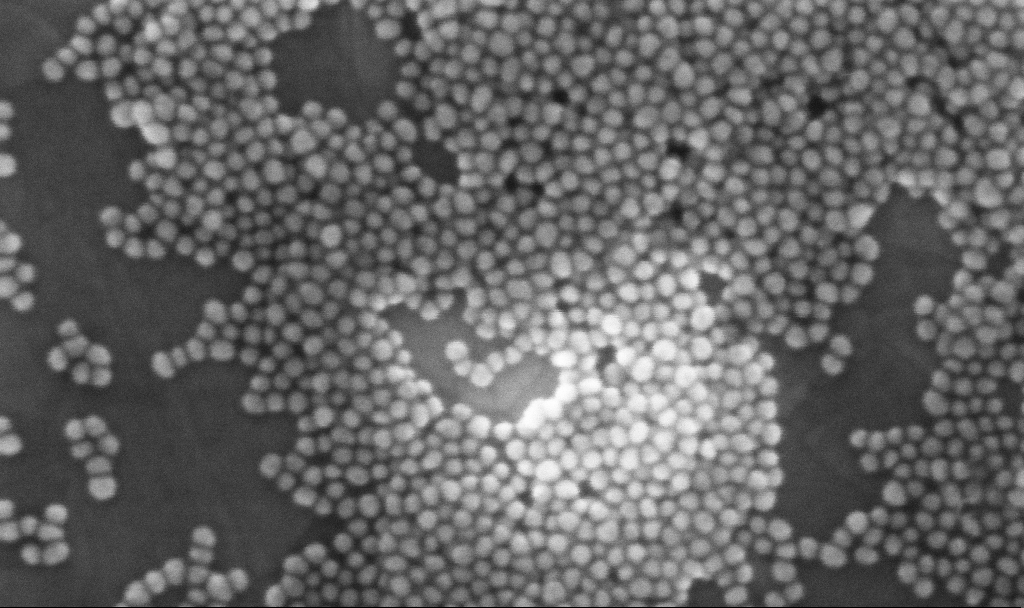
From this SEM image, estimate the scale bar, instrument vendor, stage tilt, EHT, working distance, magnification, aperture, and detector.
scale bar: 100 nm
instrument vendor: Zeiss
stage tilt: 0°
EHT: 10 kV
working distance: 3.4 mm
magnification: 448.51 K X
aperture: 30 µm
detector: InLens